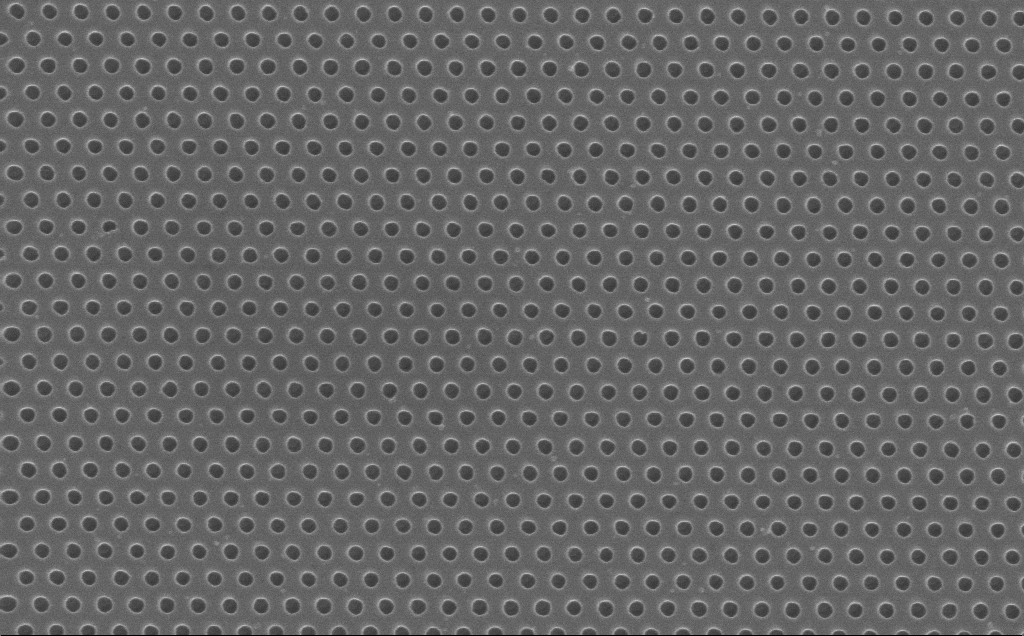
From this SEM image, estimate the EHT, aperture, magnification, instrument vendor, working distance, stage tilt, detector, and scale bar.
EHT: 10 kV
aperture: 30 µm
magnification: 57.52 K X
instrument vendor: Zeiss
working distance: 7 mm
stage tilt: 0°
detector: InLens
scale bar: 1000 nm